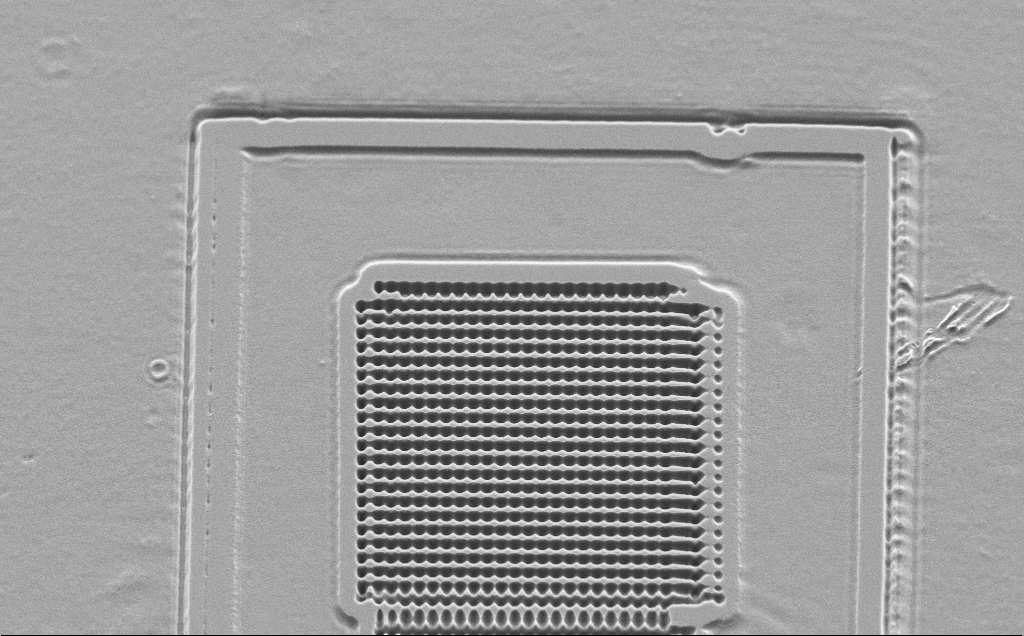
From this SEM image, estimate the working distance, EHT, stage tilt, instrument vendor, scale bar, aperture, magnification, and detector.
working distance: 10 mm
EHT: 5 kV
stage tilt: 45°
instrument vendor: Zeiss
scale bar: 20000 nm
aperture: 30 µm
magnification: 2.51 K X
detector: SE2